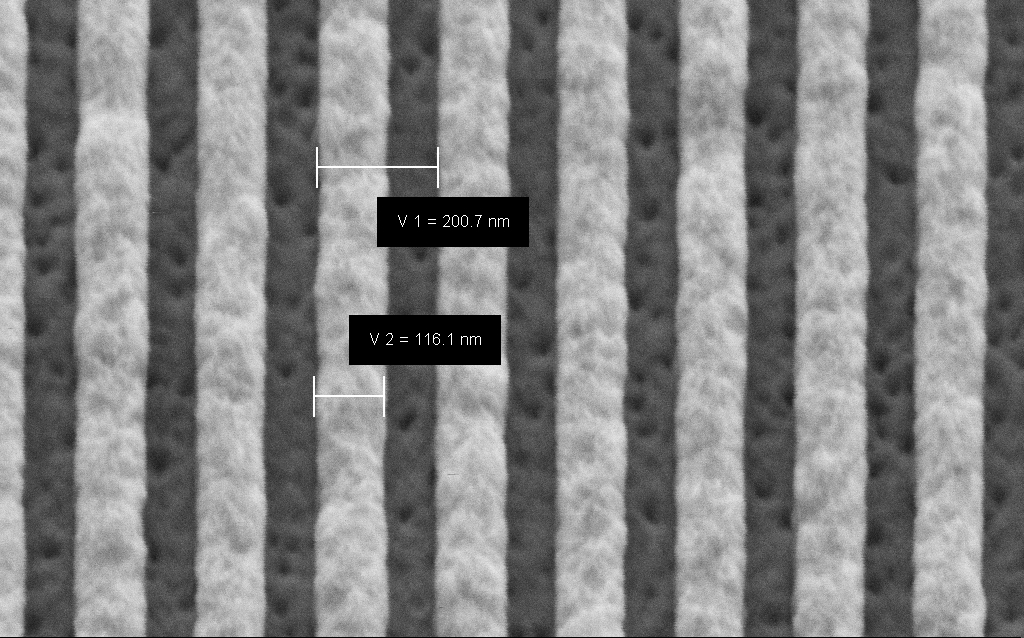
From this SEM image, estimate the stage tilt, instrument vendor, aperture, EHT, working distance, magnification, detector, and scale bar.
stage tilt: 45°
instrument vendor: Zeiss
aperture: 30 µm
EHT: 3 kV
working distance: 6.6 mm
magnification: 221.36 K X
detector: SE2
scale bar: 200 nm